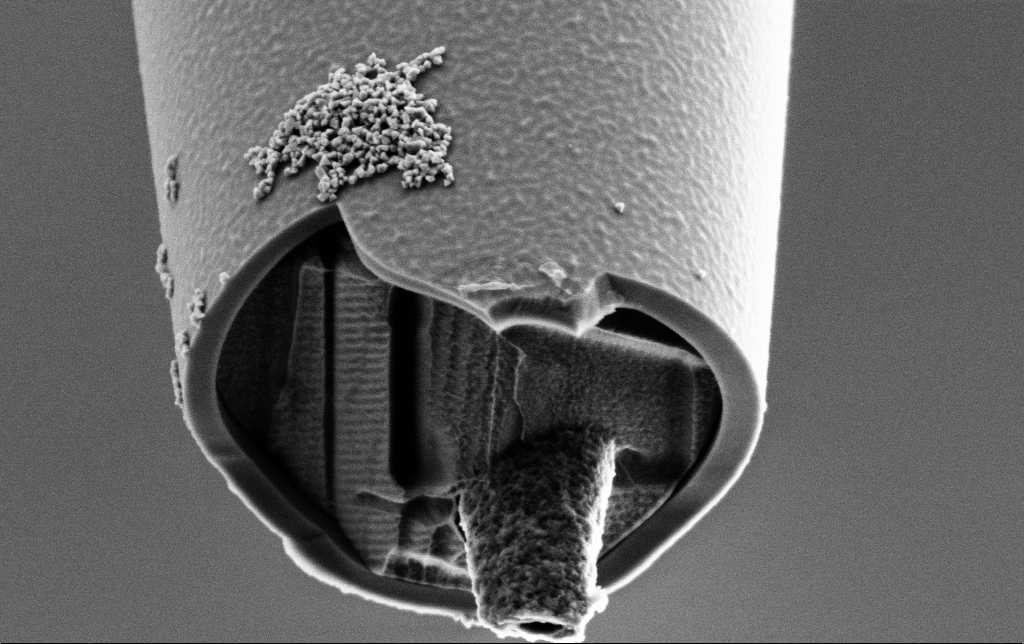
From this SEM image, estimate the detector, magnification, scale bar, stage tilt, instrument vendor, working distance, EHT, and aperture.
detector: SE2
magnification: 50 K X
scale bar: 1000 nm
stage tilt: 45°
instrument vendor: Zeiss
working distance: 7.3 mm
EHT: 2 kV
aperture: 30 µm